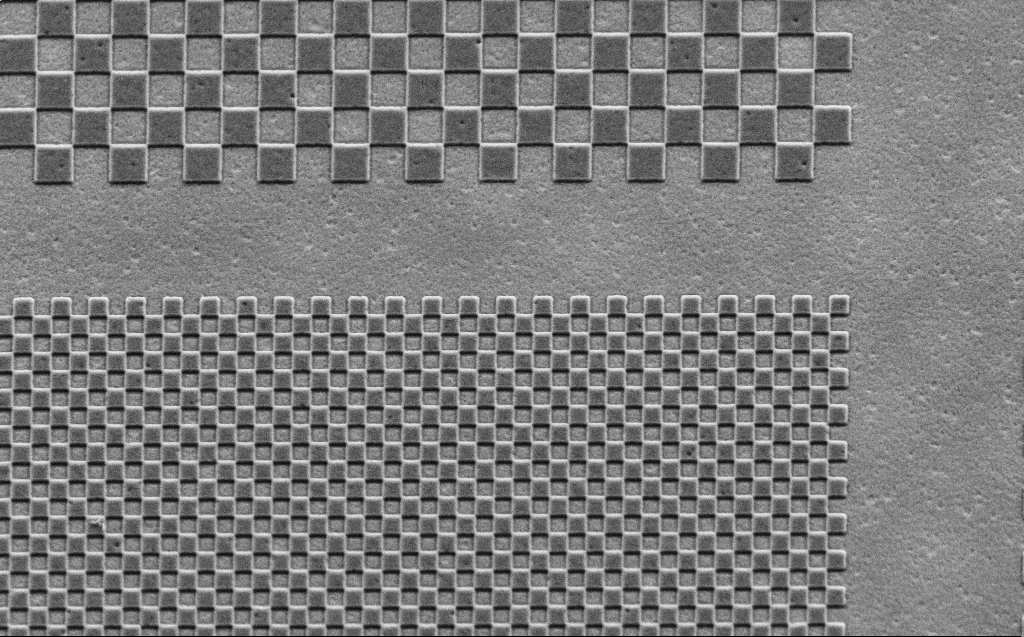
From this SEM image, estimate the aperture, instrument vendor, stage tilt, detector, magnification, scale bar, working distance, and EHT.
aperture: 30 µm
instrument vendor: Zeiss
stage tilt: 30°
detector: SE2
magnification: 13.66 K X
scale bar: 1000 nm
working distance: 5 mm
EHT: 2.5 kV